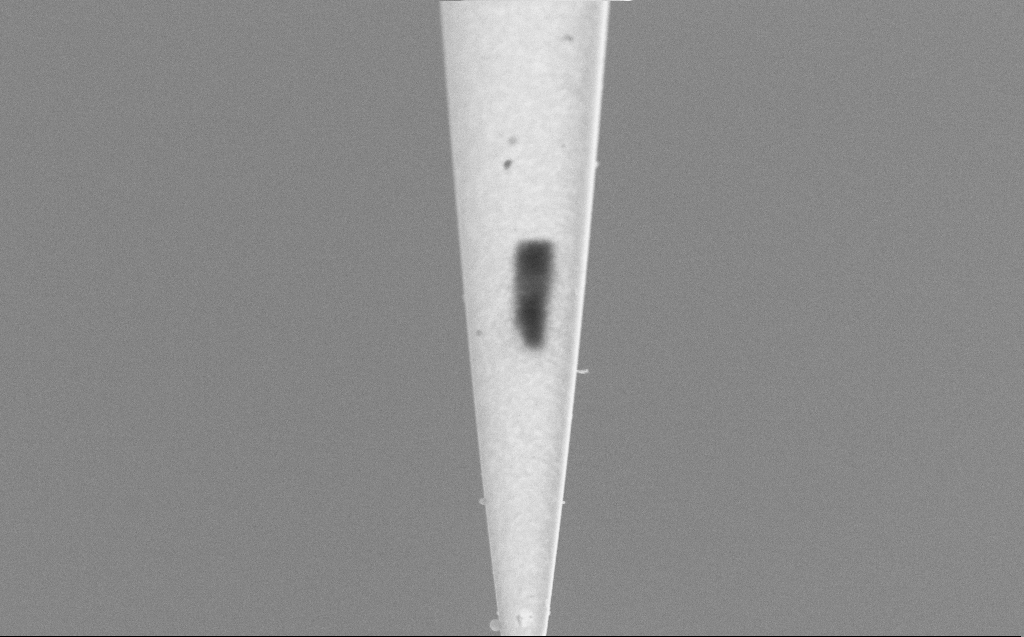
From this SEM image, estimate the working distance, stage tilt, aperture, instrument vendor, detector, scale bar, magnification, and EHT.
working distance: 4 mm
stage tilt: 45°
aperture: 30 µm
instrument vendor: Zeiss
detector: SE2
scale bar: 2000 nm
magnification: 26.74 K X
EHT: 5 kV